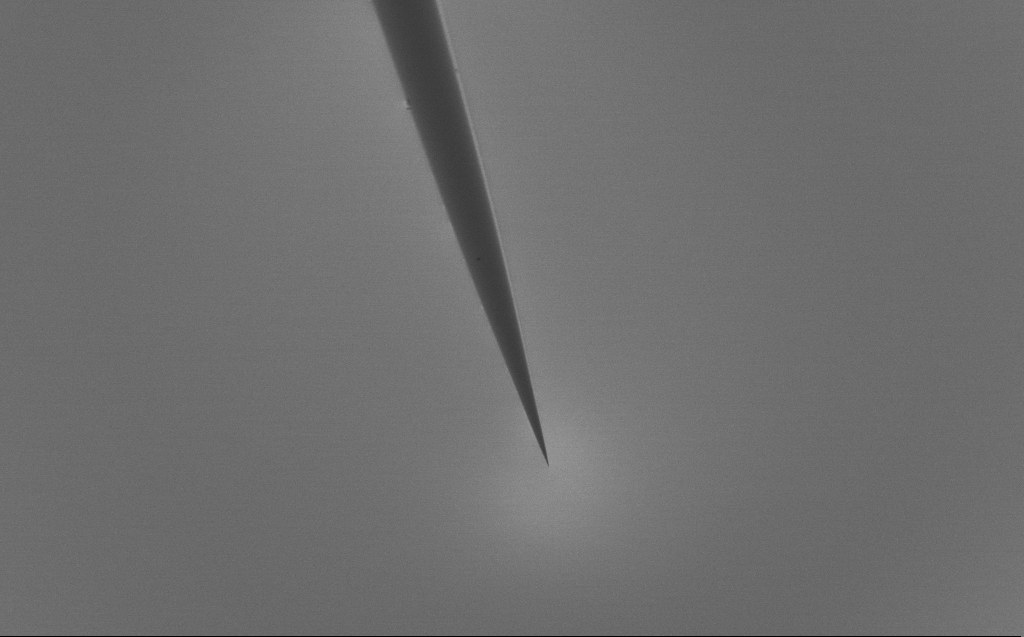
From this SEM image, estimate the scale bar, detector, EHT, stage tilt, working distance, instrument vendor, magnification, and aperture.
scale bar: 20000 nm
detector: SE2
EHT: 0.8 kV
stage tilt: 39.3°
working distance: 5 mm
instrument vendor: Zeiss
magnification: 1 K X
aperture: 30 µm